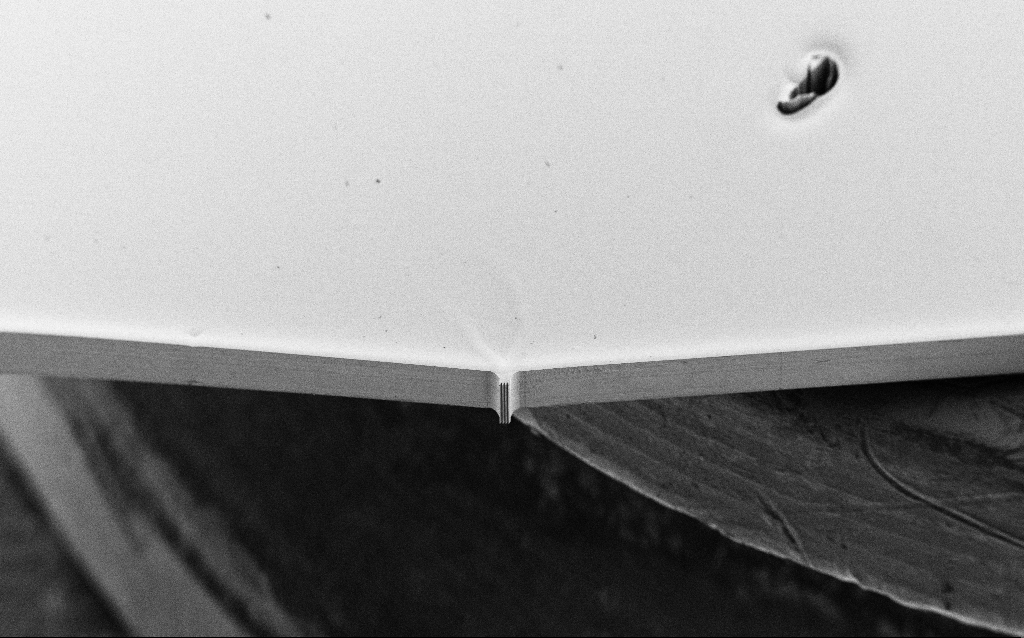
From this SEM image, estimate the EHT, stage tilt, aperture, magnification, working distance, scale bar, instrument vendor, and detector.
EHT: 5 kV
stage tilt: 45°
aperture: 30 µm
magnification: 0.099 K X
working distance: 6 mm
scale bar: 200000 nm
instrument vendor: Zeiss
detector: SE2